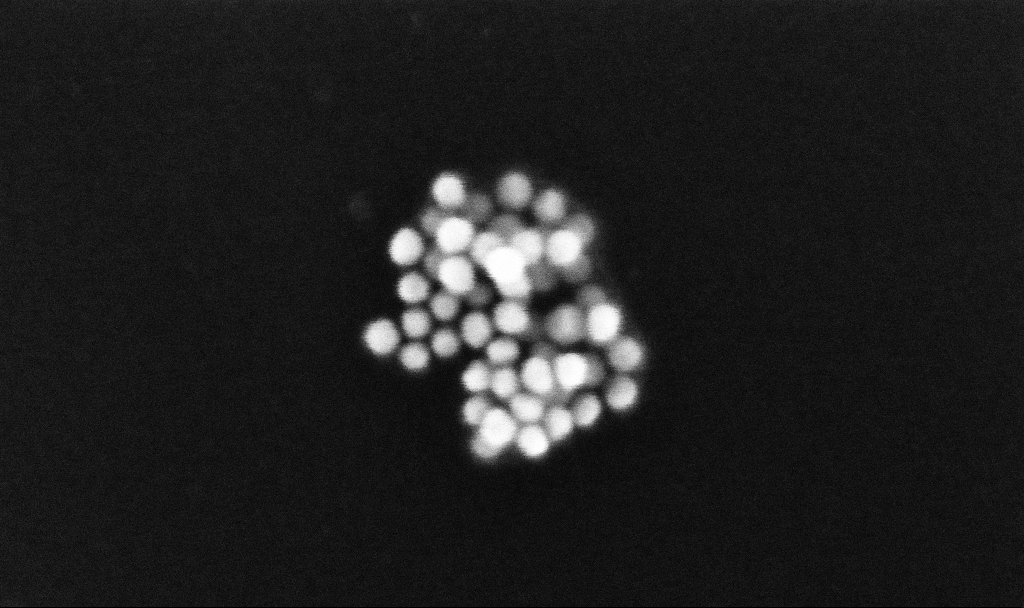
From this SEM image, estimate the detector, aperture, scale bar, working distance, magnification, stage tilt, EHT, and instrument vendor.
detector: InLens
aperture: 30 µm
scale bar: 100 nm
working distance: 3.3 mm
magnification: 616.96 K X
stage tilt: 0°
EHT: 10 kV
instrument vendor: Zeiss